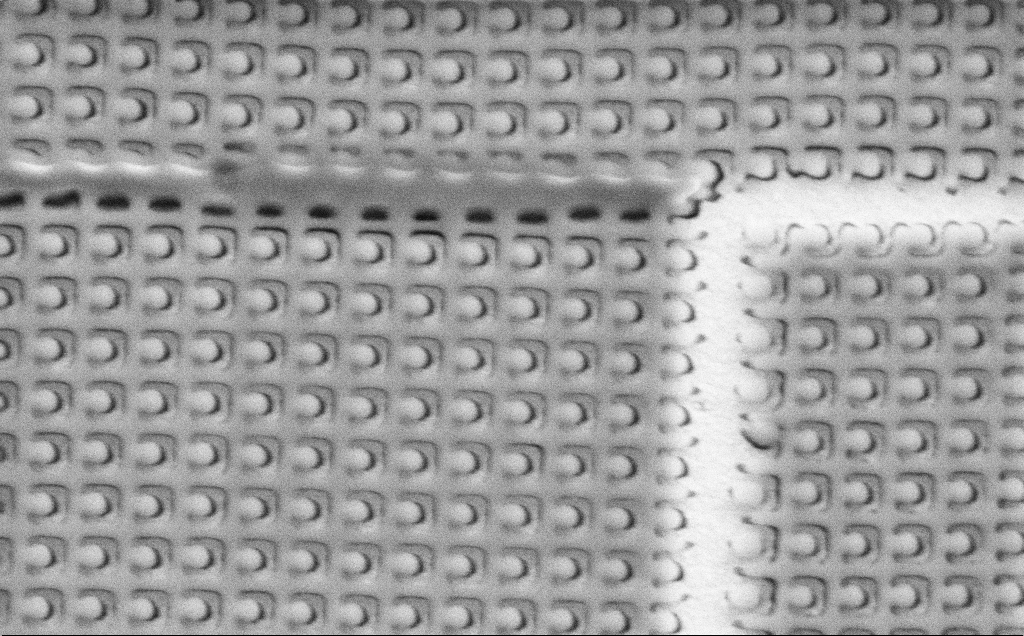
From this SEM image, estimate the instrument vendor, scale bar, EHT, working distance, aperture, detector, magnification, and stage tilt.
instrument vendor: Zeiss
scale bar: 1000 nm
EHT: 3 kV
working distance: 7.9 mm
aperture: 30 µm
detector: SE2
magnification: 41.48 K X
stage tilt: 0°